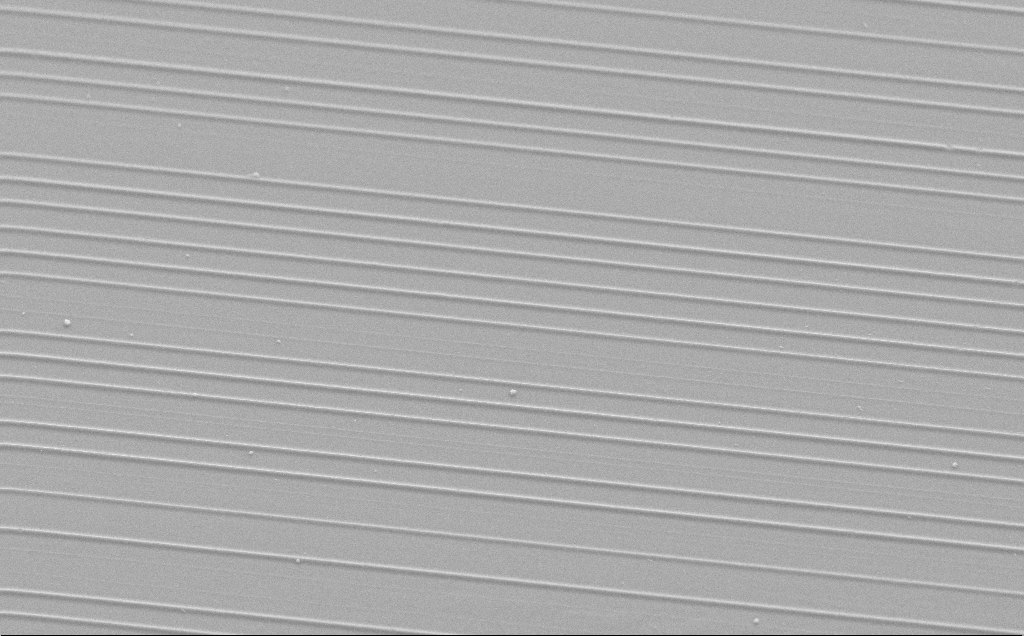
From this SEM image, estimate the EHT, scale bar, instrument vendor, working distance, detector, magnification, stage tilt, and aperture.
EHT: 10 kV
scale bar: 10000 nm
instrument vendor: Zeiss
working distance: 6 mm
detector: SE2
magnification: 1.31 K X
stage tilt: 45°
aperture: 30 µm